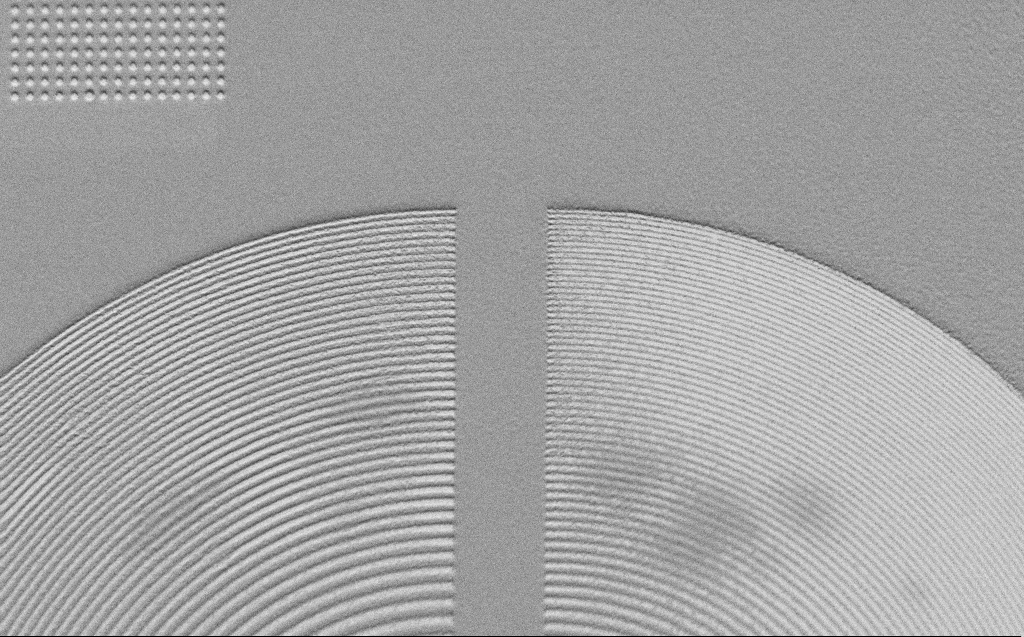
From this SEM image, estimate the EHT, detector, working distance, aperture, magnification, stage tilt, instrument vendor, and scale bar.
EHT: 1 kV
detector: SE2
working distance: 5 mm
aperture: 30 µm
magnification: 3.4 K X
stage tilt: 45°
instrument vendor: Zeiss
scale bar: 10000 nm